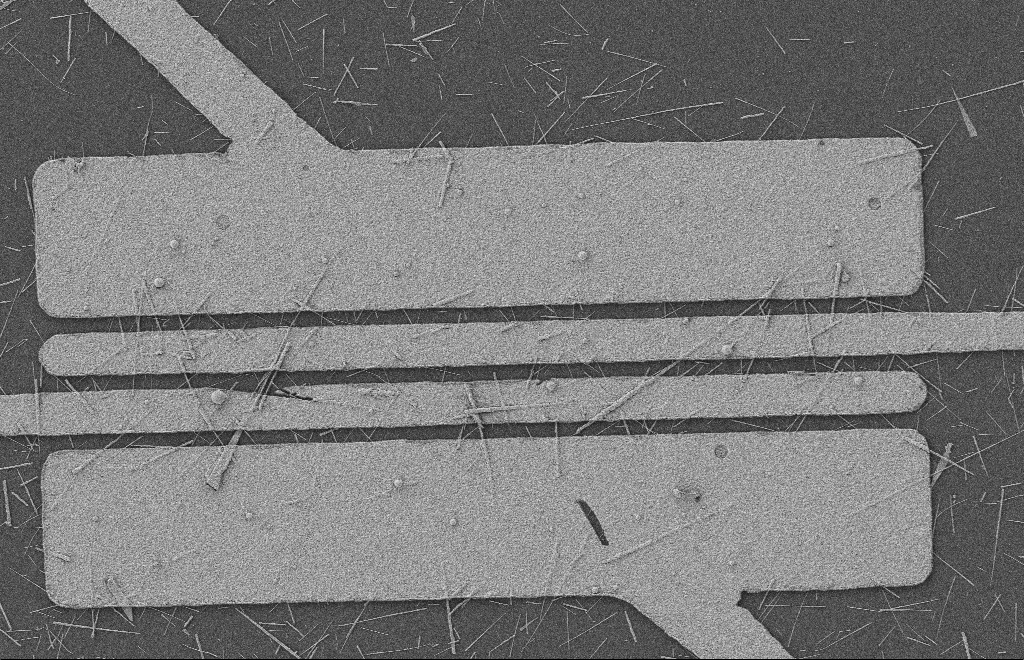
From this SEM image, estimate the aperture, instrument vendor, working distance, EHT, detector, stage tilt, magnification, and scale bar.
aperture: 20 µm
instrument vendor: Zeiss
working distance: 8 mm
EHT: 2 kV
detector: SE2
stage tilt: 0°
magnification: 5.33 K X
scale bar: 2000 nm